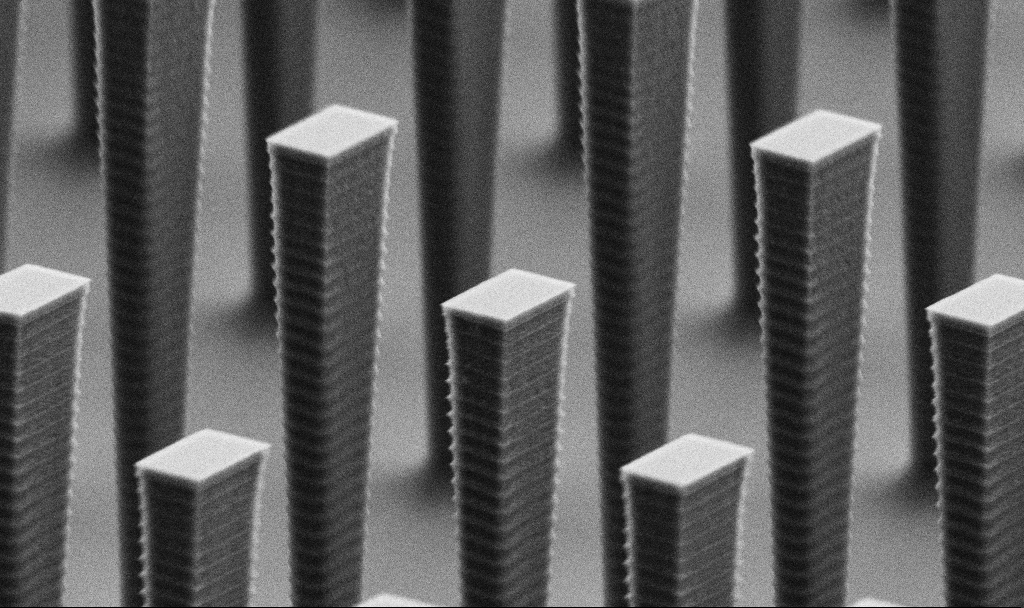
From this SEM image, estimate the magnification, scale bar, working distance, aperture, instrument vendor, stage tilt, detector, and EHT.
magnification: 16.43 K X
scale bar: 1000 nm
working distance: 6.3 mm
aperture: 30 µm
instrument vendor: Zeiss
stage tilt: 70°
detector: SE2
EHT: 5 kV